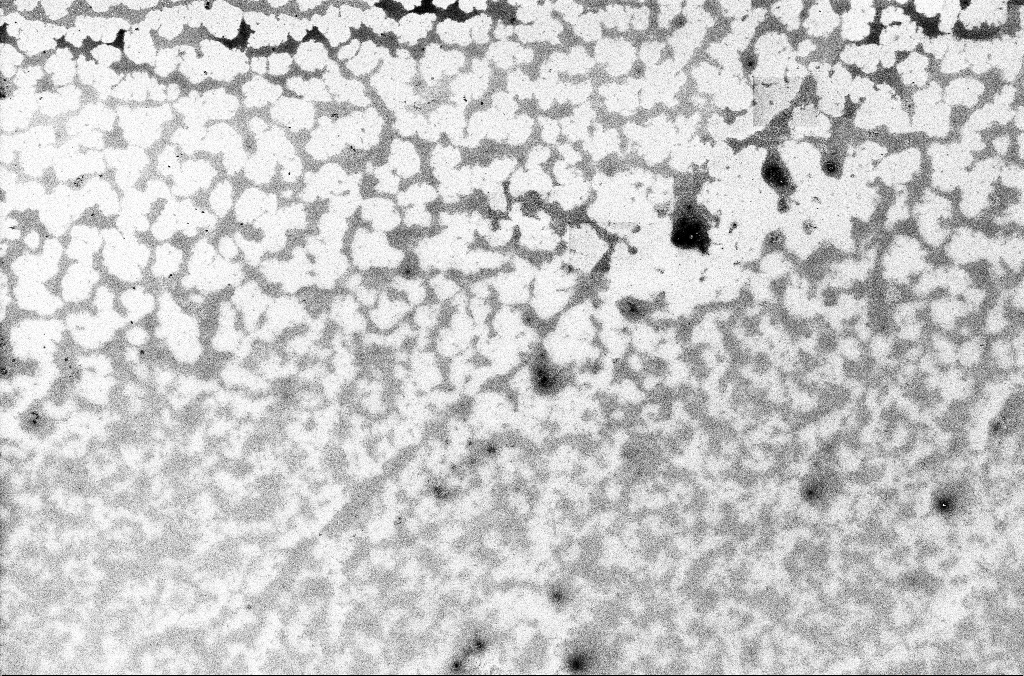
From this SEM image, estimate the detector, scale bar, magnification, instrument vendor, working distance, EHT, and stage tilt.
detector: InLens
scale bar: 10000 nm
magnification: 1.75 K X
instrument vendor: Zeiss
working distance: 3.6 mm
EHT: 2 kV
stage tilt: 0°